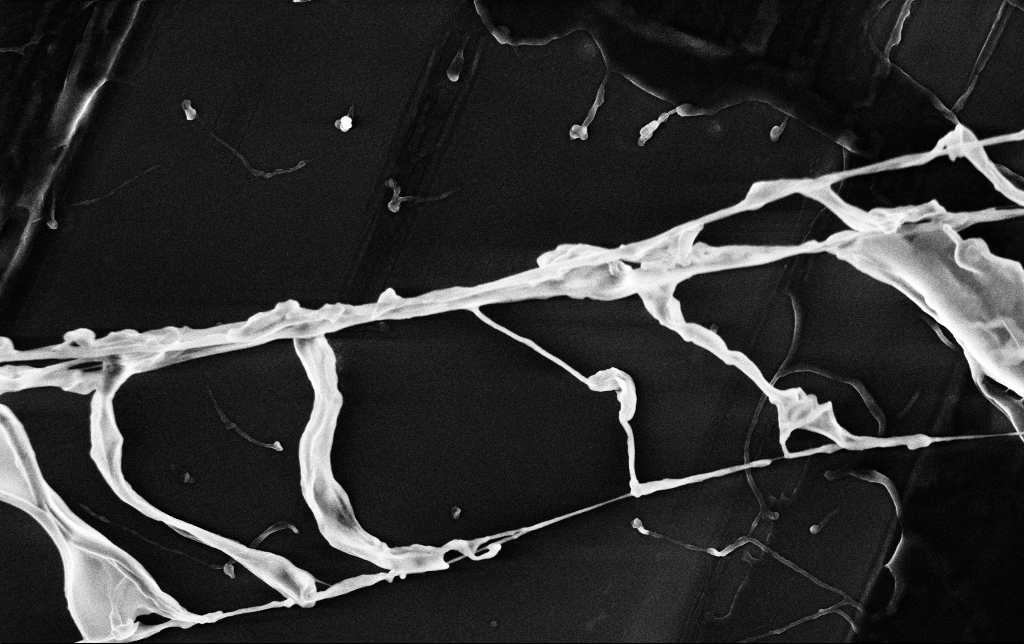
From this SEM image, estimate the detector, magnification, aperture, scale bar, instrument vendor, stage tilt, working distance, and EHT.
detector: InLens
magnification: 23.28 K X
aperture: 30 µm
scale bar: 1000 nm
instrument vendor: Zeiss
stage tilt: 0°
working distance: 3.5 mm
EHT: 3 kV